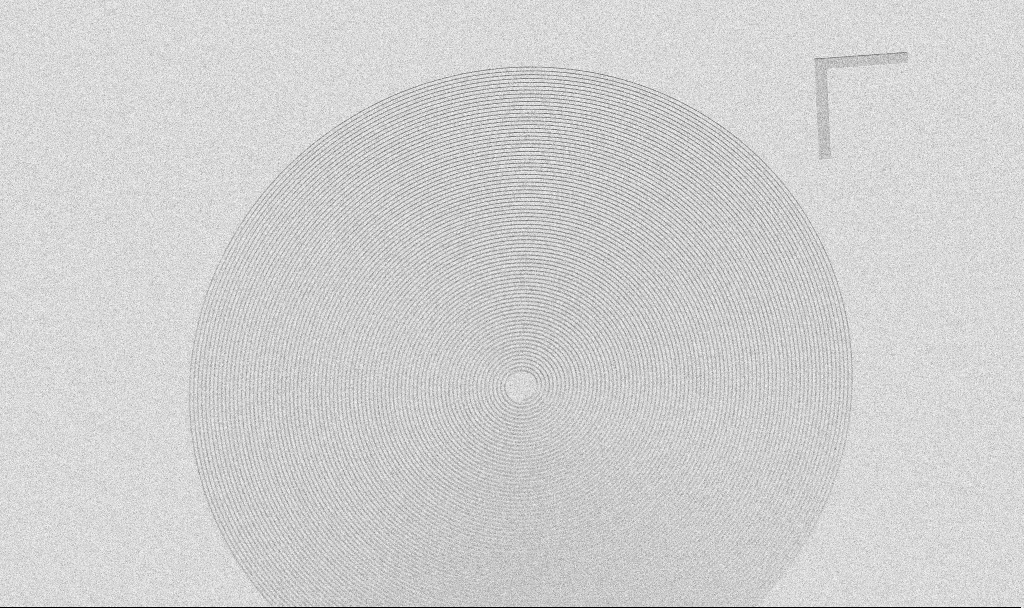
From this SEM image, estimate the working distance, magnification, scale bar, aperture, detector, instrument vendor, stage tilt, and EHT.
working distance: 9.5 mm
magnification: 2.29 K X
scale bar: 20000 nm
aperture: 30 µm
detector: SE2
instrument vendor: Zeiss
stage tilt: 45°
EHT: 5 kV